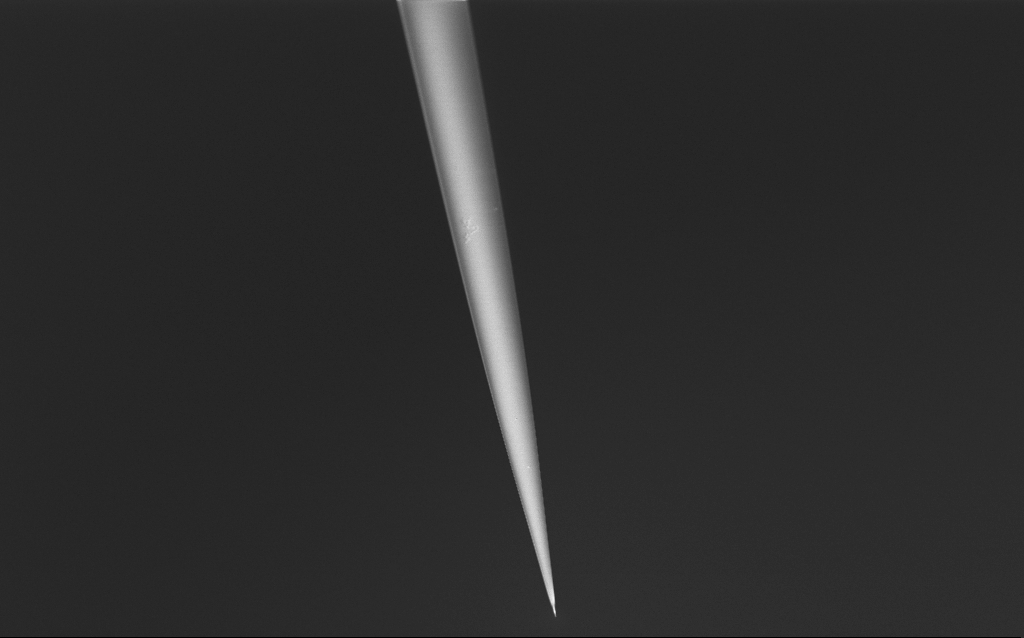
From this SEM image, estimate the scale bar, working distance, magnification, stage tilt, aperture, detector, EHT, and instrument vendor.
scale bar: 20000 nm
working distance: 6 mm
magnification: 1 K X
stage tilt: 45°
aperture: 30 µm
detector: InLens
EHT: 1.5 kV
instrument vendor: Zeiss